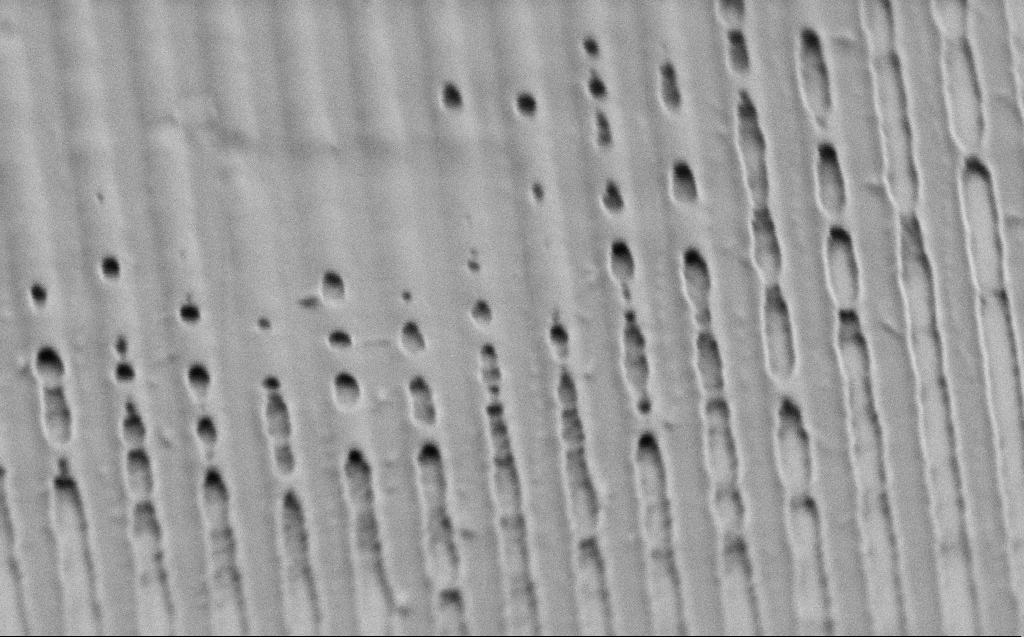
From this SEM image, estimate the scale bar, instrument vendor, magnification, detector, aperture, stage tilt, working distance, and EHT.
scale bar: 1000 nm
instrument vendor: Zeiss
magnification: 54.51 K X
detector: SE2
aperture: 30 µm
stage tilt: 60.7°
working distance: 3 mm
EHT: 1 kV